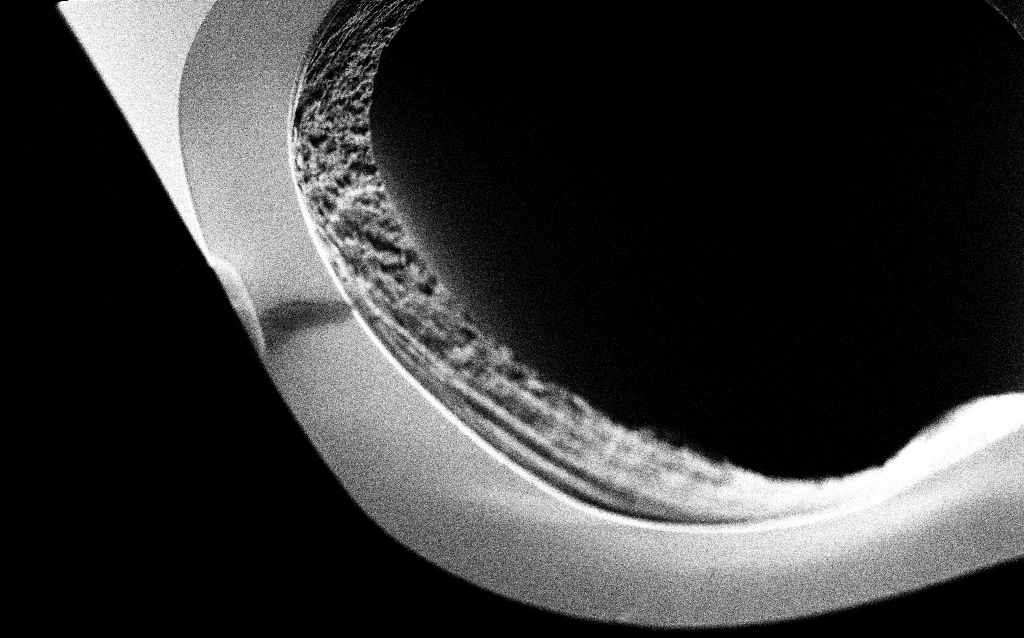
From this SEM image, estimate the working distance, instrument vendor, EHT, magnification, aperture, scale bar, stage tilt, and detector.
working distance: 6.4 mm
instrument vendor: Zeiss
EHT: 1 kV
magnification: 10 K X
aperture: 30 µm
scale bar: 2000 nm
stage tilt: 45°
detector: SE2